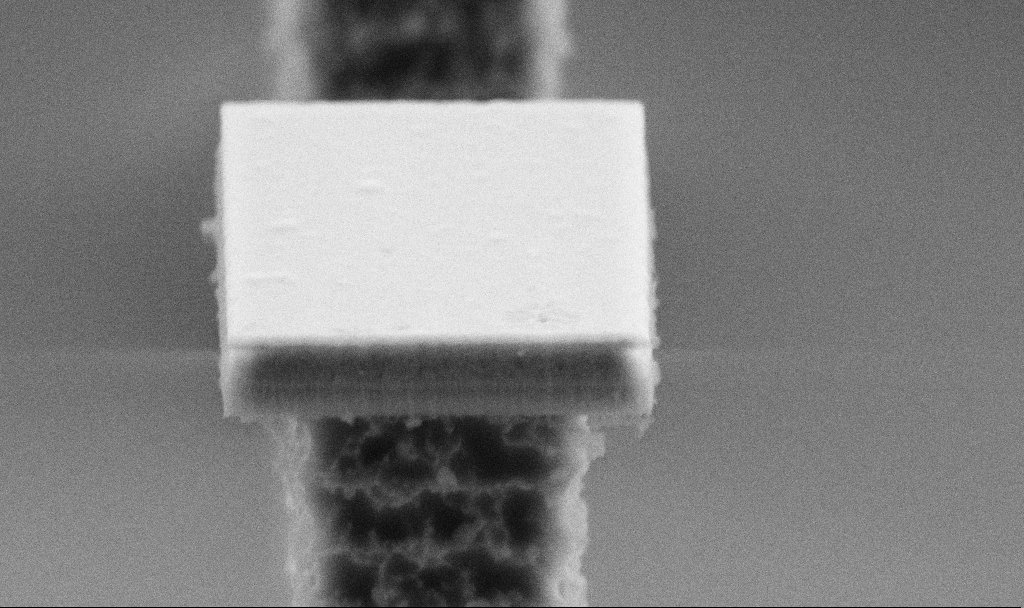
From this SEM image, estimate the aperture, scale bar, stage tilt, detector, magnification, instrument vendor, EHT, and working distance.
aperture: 20 µm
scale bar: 1000 nm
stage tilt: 70°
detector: SE2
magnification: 52.64 K X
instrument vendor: Zeiss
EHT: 5 kV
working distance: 5 mm